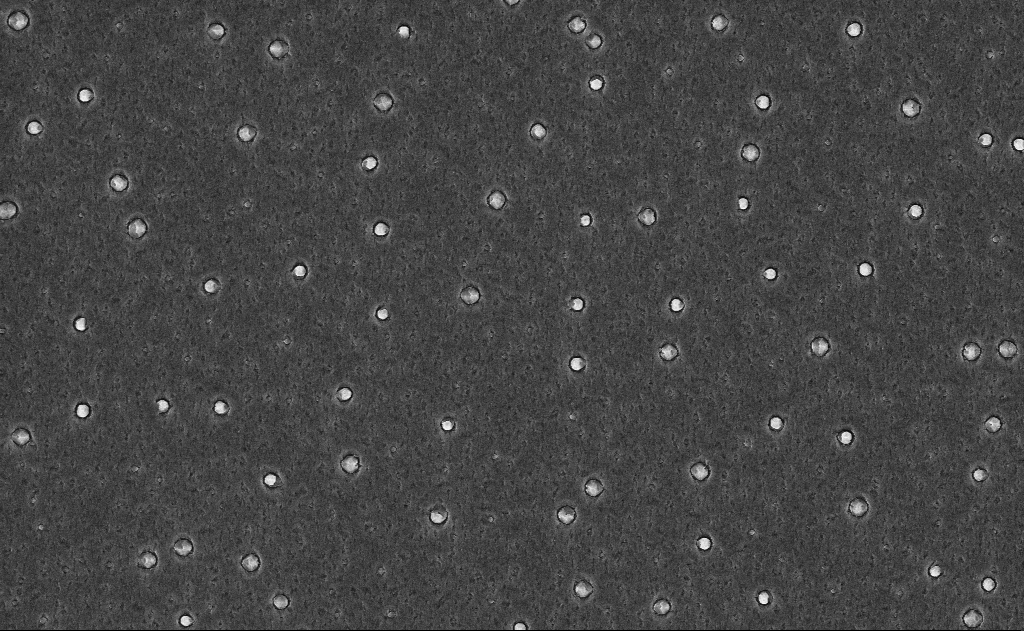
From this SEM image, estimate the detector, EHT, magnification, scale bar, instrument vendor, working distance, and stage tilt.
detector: InLens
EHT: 10 kV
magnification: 10 K X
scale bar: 2000 nm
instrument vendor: Zeiss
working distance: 18 mm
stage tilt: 0°